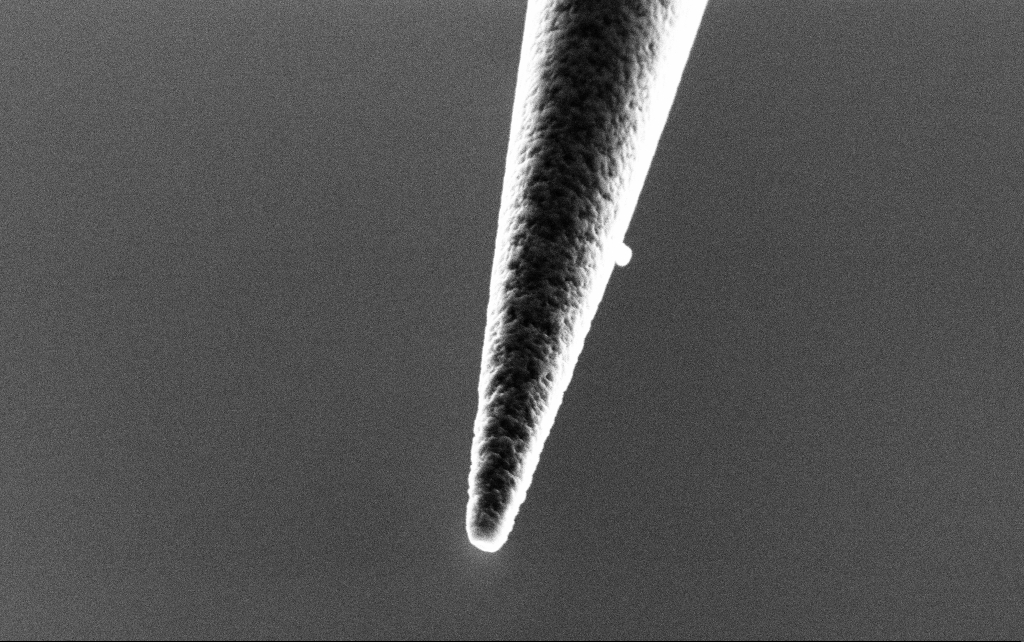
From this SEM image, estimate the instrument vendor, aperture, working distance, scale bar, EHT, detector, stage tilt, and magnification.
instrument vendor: Zeiss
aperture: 30 µm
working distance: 7.4 mm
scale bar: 1000 nm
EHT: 3 kV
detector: SE2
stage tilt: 45°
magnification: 50 K X